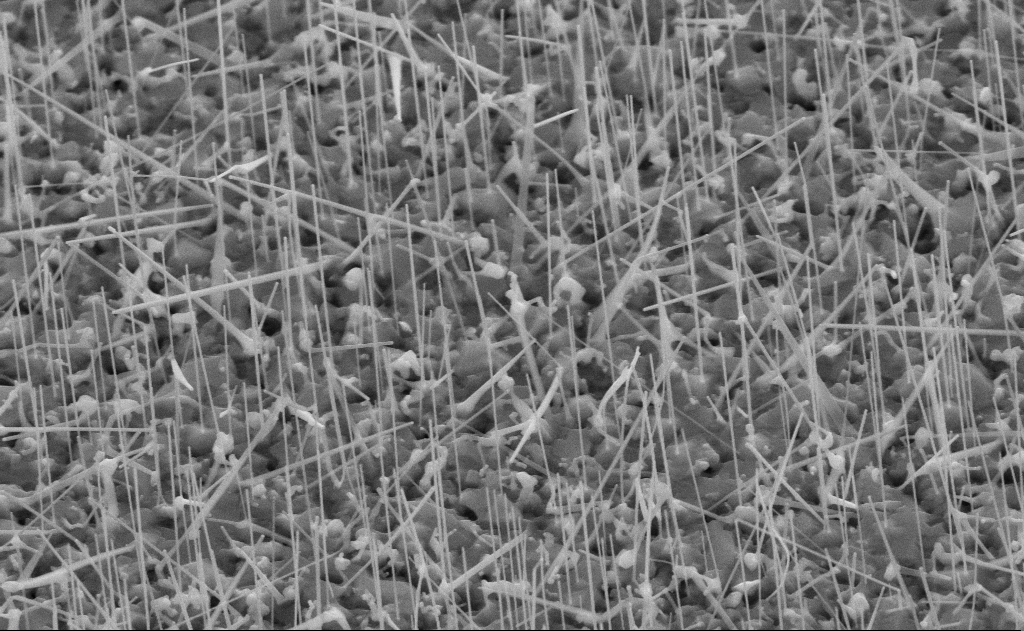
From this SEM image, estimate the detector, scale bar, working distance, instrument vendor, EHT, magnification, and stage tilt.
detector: SE2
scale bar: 1000 nm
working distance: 11 mm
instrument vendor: Zeiss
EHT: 10 kV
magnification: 40 K X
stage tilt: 45°